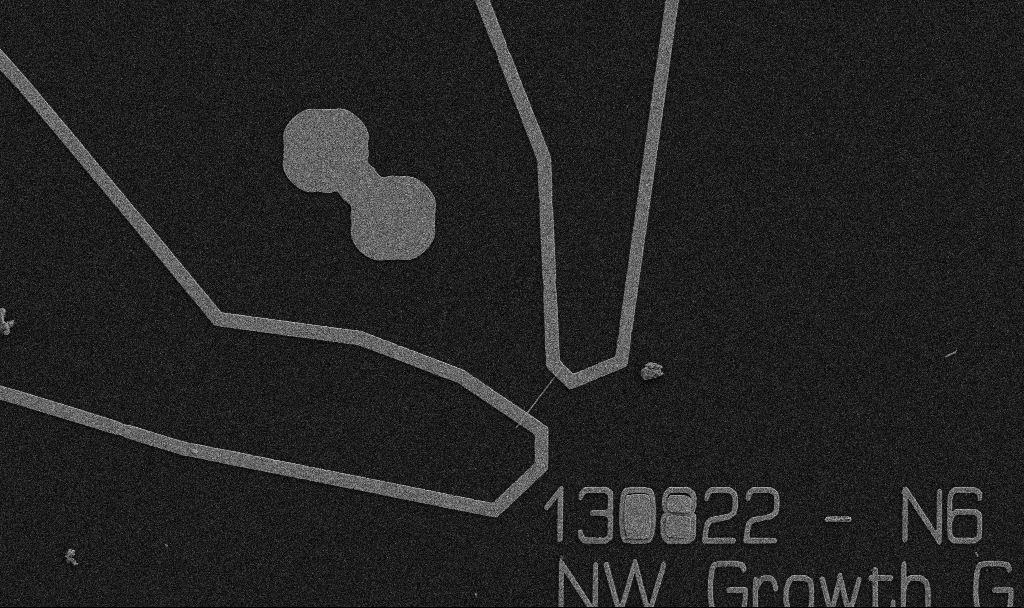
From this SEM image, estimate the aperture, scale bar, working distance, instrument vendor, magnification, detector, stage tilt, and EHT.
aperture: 30 µm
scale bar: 10000 nm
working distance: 10.7 mm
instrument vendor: Zeiss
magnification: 5 K X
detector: SE2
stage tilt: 0°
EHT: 5 kV